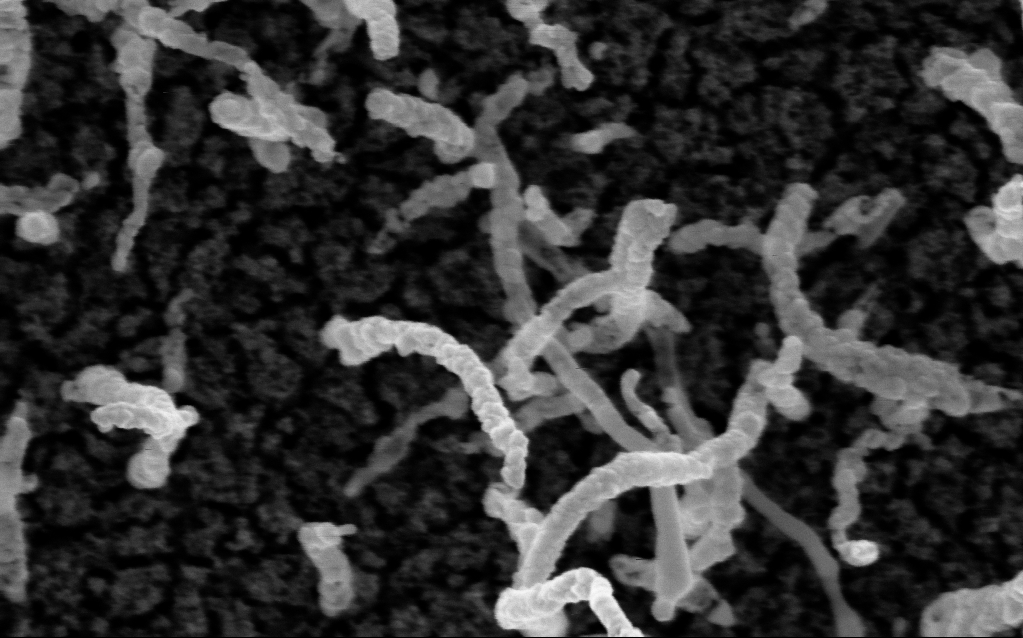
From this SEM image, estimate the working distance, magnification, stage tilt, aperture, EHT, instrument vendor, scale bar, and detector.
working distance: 2.1 mm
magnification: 200 K X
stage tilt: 0°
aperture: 30 µm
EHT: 5 kV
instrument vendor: Zeiss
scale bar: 100 nm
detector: InLens